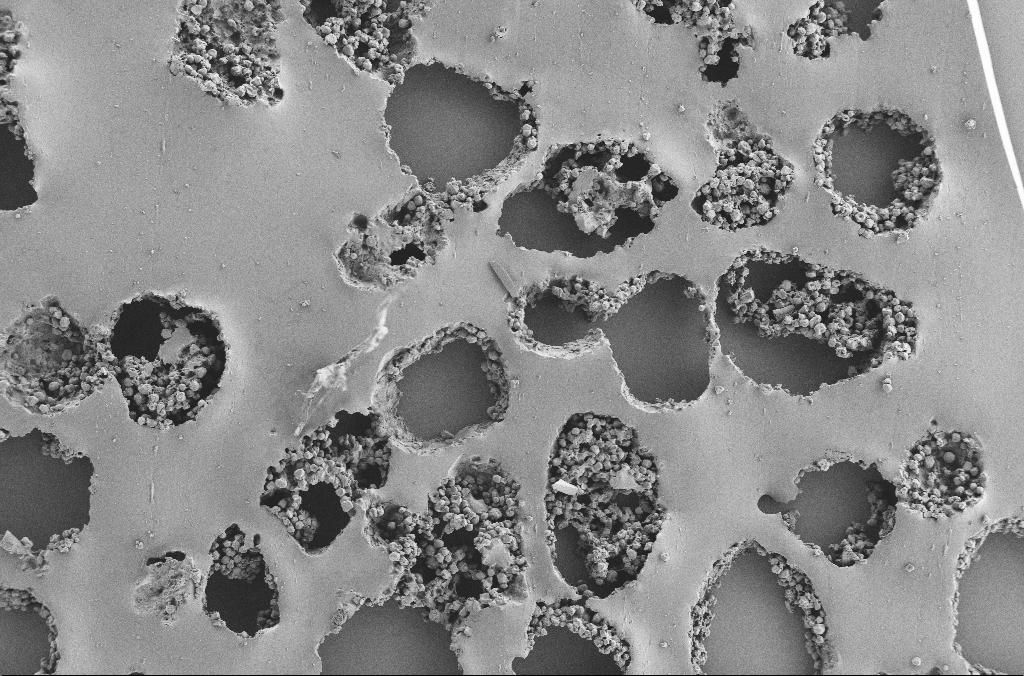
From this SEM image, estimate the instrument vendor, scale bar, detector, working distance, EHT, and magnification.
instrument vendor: Zeiss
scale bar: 100000 nm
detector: SE2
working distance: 3.7 mm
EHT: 2 kV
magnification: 0.25 K X